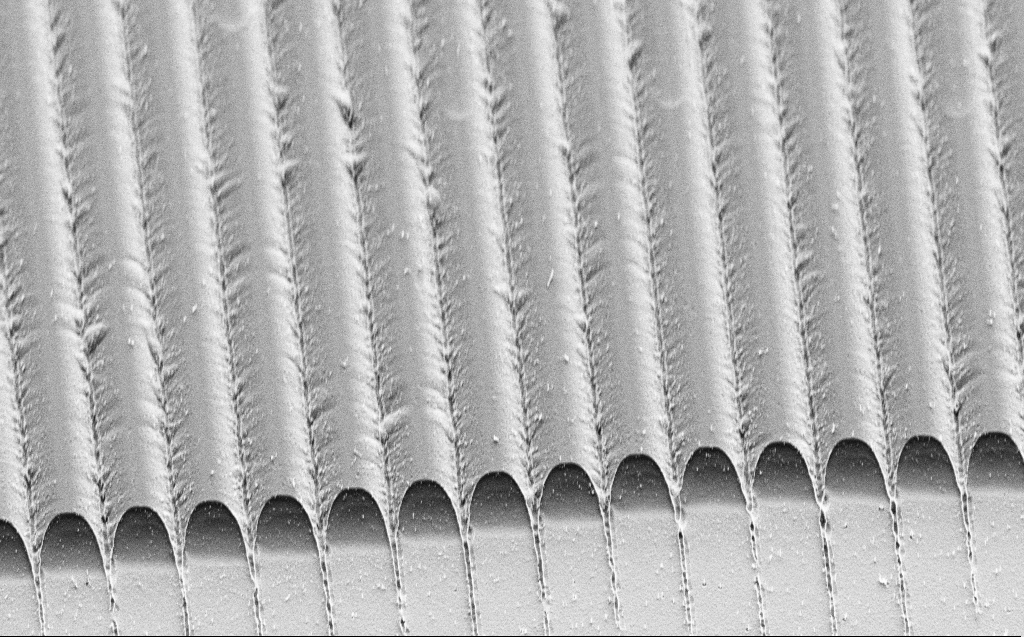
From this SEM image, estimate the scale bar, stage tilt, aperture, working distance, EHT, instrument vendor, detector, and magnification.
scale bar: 20000 nm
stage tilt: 45°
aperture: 30 µm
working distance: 9 mm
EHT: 3 kV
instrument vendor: Zeiss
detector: SE2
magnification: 2.63 K X